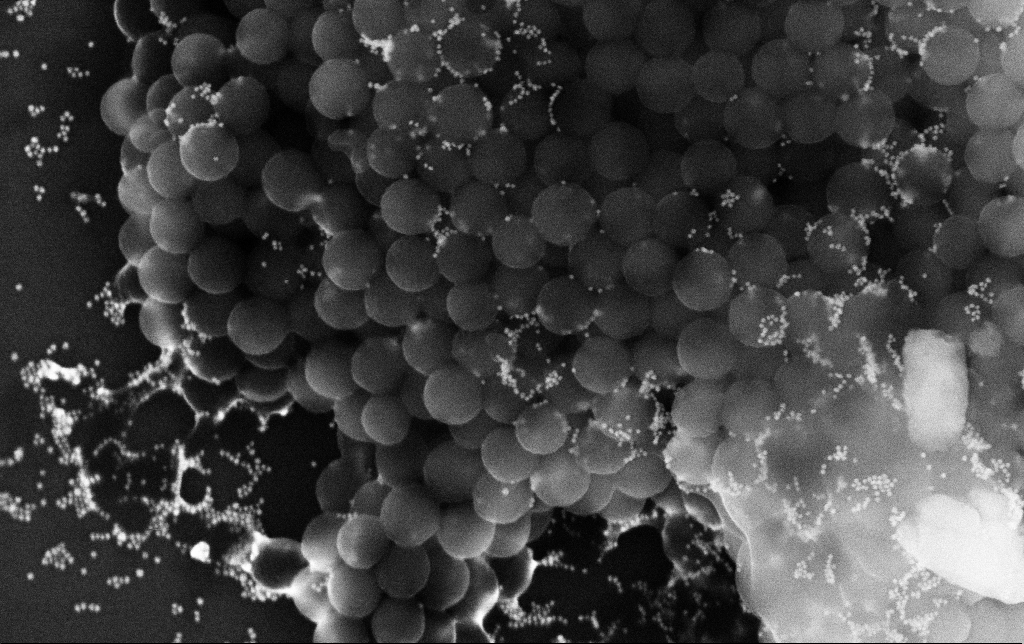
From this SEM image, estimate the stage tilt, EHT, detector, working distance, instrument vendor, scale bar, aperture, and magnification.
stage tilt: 0°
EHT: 10 kV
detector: InLens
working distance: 3.1 mm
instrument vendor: Zeiss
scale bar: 200 nm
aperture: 30 µm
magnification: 147.96 K X